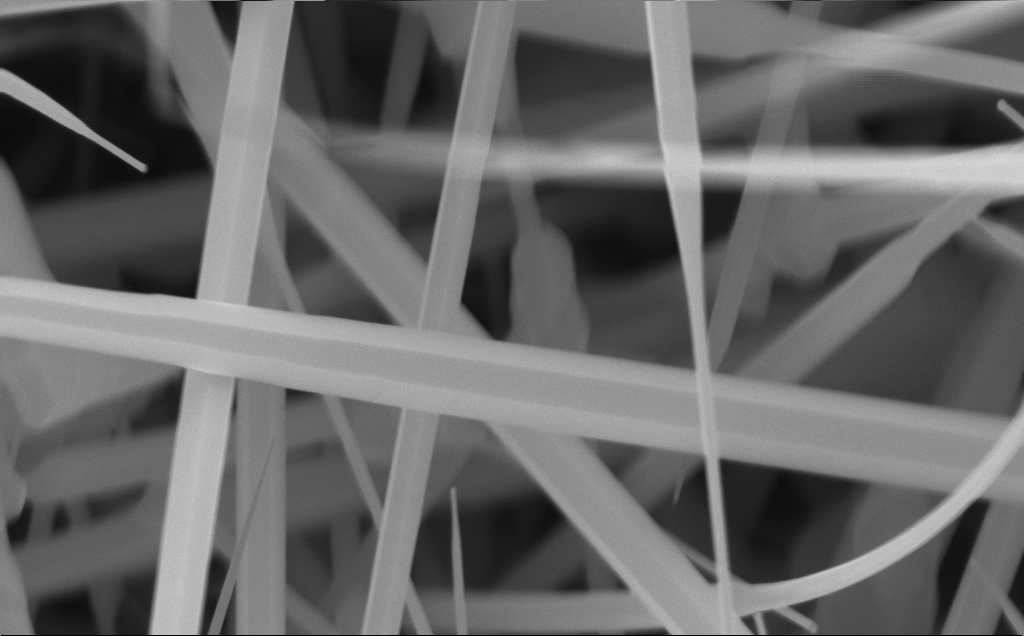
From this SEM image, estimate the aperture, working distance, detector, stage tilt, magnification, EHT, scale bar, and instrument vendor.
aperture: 30 µm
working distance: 4 mm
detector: InLens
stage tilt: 0°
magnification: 80 K X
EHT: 10 kV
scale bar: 200 nm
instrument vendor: Zeiss